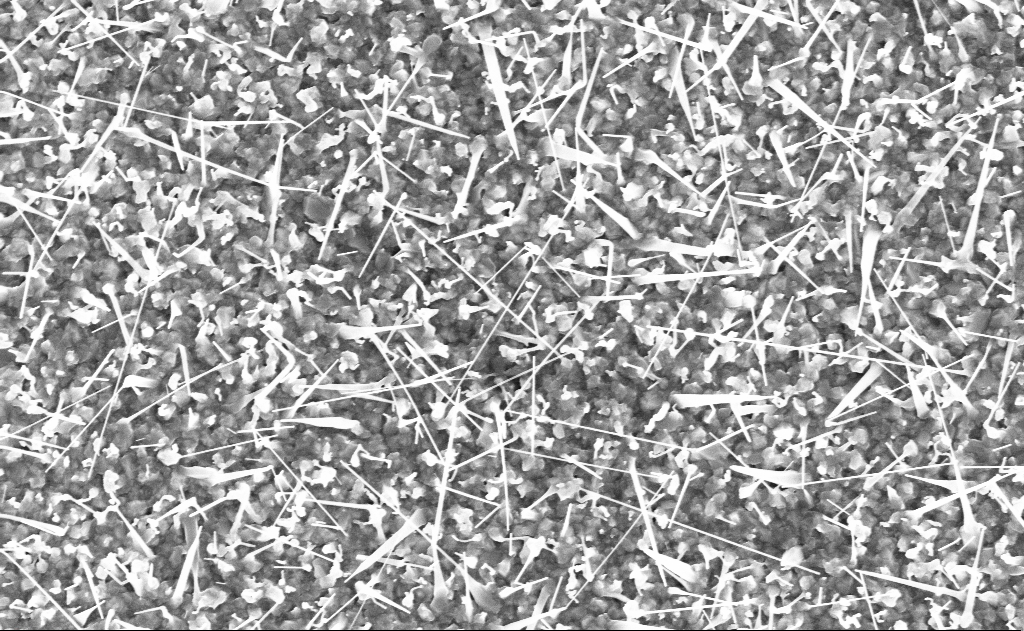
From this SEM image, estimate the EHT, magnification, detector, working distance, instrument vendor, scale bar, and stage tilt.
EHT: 10 kV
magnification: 20 K X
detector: InLens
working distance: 12 mm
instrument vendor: Zeiss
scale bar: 2000 nm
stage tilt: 0°